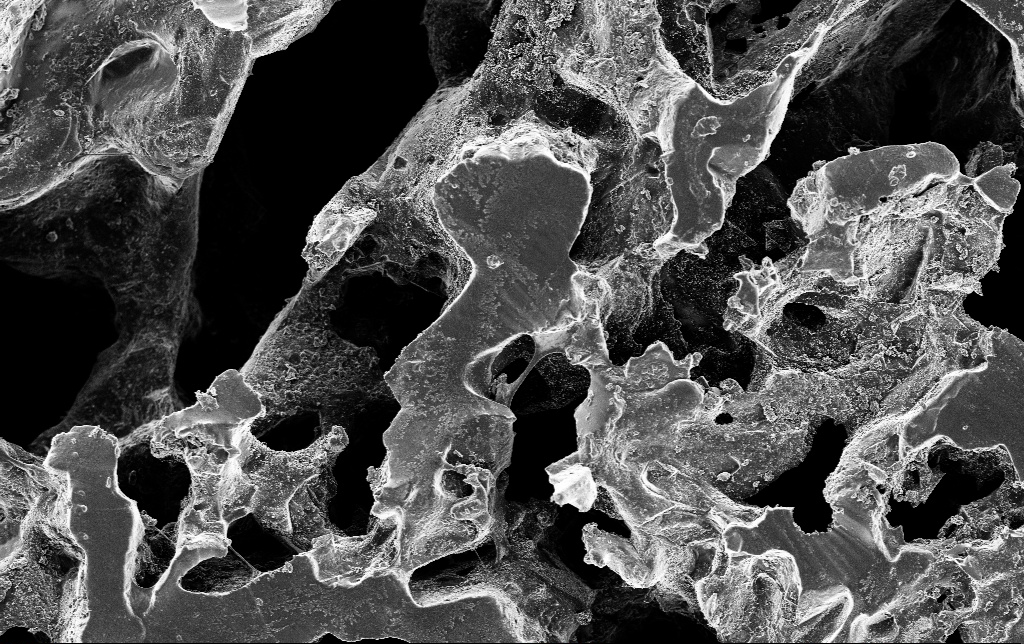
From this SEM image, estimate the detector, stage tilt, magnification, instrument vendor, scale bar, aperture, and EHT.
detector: InLens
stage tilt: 0°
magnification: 1 K X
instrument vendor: Zeiss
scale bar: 20000 nm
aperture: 30 µm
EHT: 3 kV